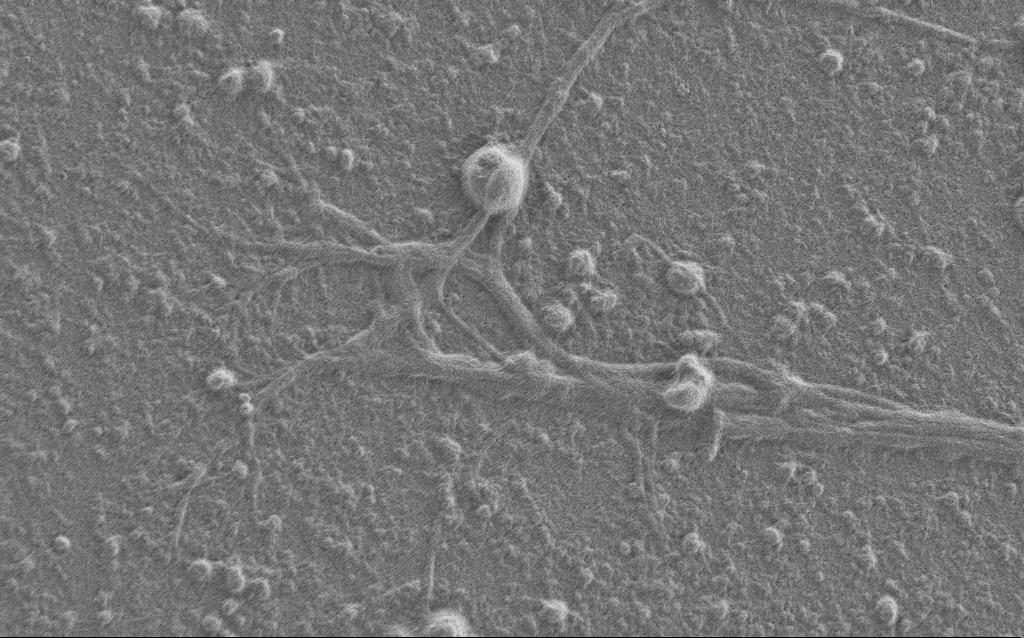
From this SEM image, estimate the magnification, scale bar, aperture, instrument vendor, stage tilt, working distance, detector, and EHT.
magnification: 5 K X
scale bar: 10000 nm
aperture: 30 µm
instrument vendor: Zeiss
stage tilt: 0°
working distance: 6 mm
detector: SE2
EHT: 1 kV